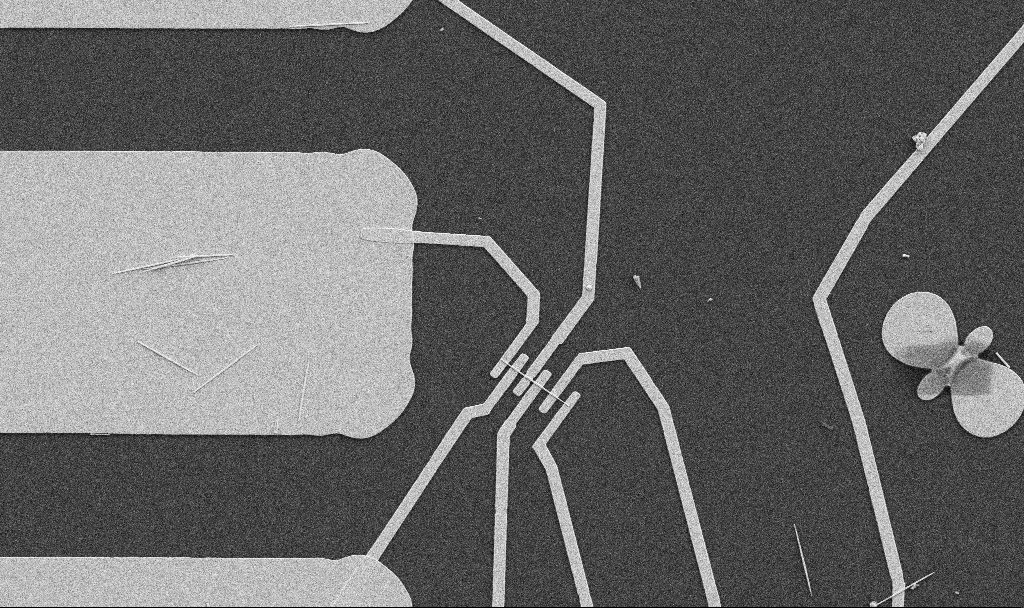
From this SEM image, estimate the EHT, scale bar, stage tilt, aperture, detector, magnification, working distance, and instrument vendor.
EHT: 5 kV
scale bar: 10000 nm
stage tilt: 0°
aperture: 30 µm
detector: SE2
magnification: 5 K X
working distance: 10.7 mm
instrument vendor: Zeiss